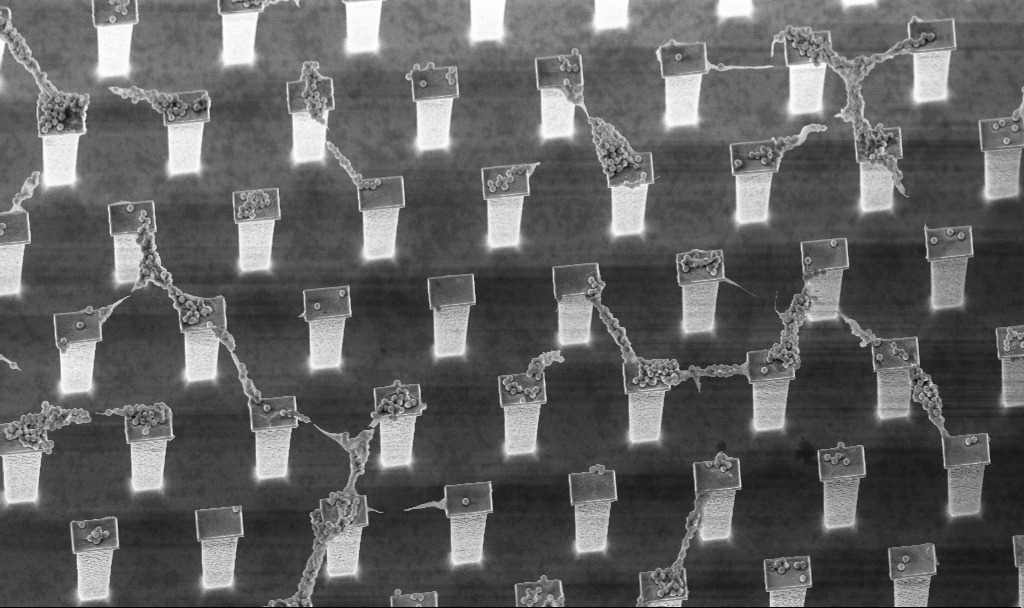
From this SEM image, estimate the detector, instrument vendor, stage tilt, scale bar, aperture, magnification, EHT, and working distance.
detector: InLens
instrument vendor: Zeiss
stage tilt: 20°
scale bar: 10000 nm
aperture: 30 µm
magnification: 3.82 K X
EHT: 5 kV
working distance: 3.2 mm